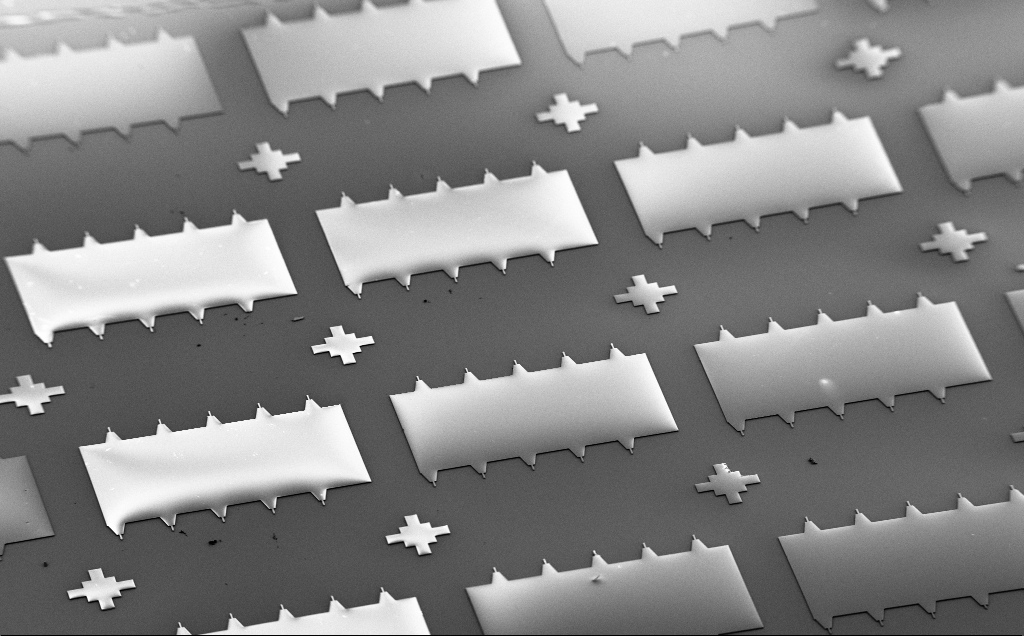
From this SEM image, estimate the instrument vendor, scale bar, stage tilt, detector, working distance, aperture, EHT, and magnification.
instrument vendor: Zeiss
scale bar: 200000 nm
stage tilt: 50°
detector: SE2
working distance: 10 mm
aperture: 30 µm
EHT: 5 kV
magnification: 0.116 K X